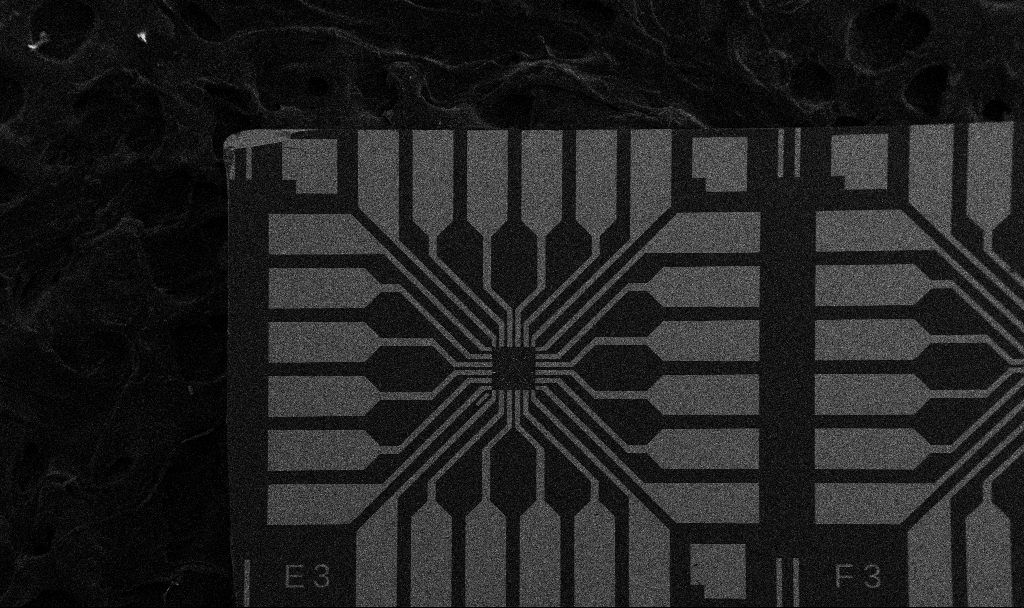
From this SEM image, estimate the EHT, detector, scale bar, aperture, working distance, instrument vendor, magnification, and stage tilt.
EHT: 5 kV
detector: SE2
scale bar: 200000 nm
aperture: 30 µm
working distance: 10.7 mm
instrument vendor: Zeiss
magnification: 0.1 K X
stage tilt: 0°